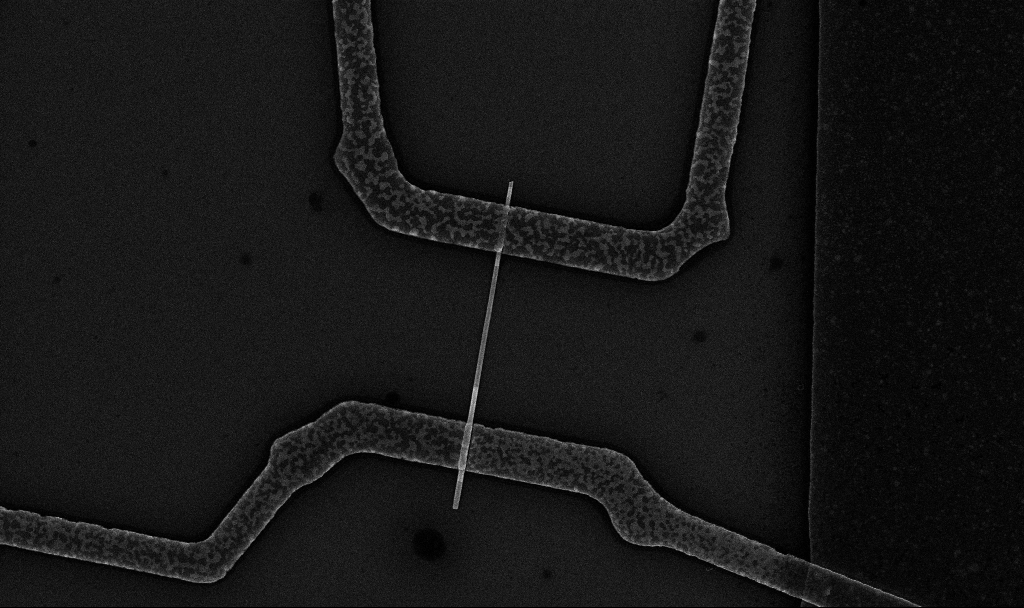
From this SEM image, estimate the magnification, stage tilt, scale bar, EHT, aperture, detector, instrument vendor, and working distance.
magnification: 19.51 K X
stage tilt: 0°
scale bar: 2000 nm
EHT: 10 kV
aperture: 30 µm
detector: InLens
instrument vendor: Zeiss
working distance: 6.7 mm